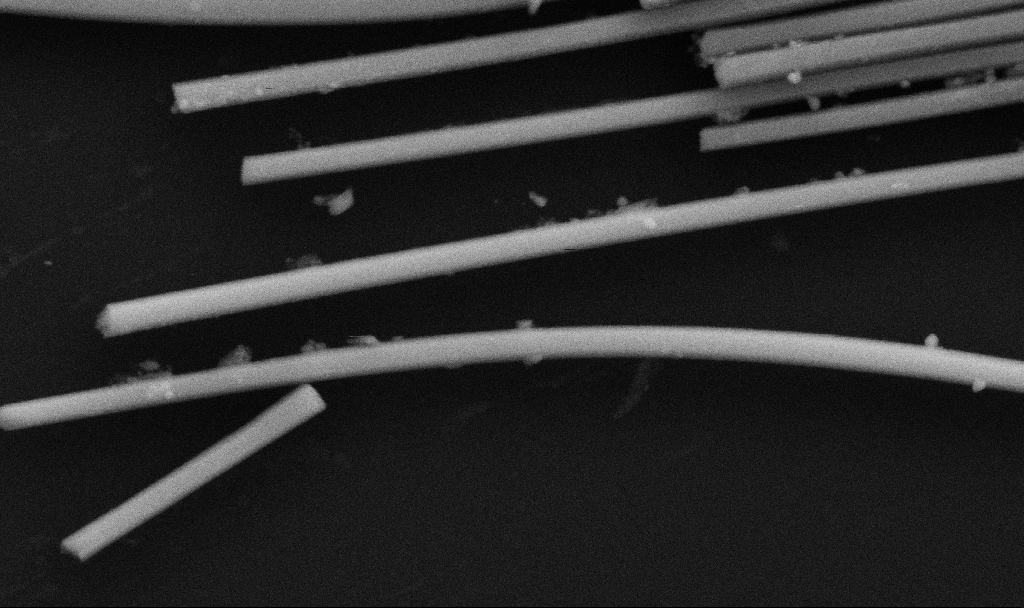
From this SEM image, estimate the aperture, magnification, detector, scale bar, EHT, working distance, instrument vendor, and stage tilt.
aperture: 30 µm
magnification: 118.35 K X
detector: SE2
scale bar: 200 nm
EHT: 5 kV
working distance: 6.7 mm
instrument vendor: Zeiss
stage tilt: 0°